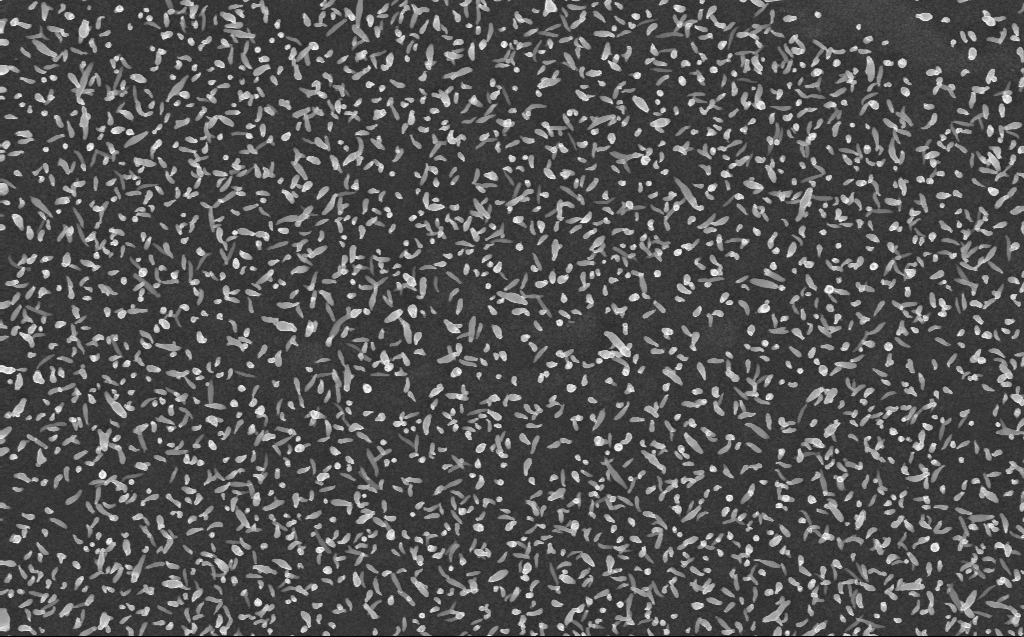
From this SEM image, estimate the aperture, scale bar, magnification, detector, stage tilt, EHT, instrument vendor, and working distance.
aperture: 30 µm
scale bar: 2000 nm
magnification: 20 K X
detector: InLens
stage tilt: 0°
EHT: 10 kV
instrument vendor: Zeiss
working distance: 3 mm